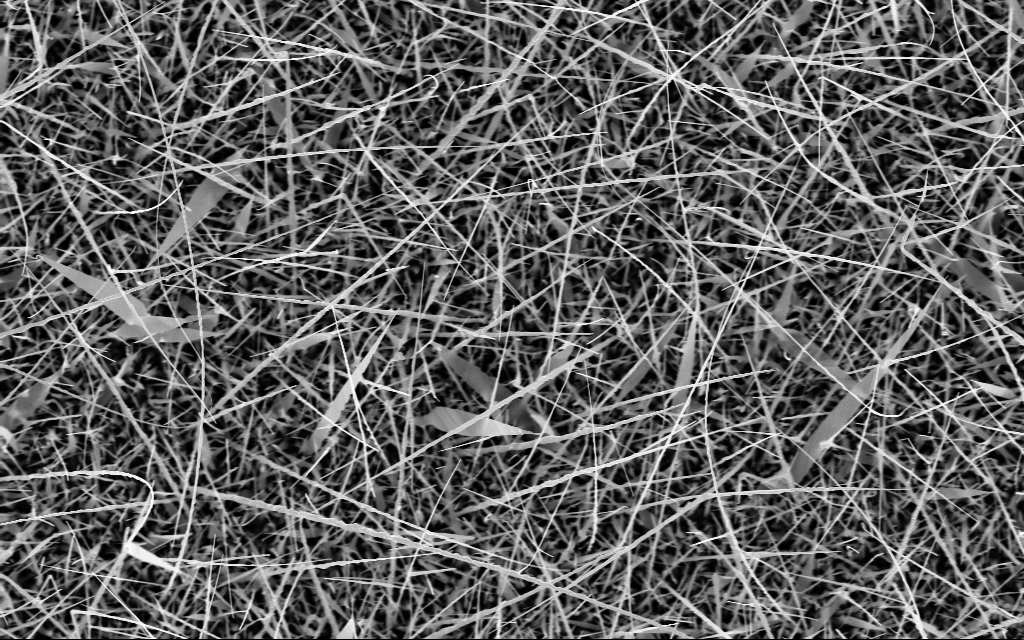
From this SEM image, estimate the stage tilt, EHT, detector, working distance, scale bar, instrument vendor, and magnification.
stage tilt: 0°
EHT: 10 kV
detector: InLens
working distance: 6 mm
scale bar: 2000 nm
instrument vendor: Zeiss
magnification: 10 K X